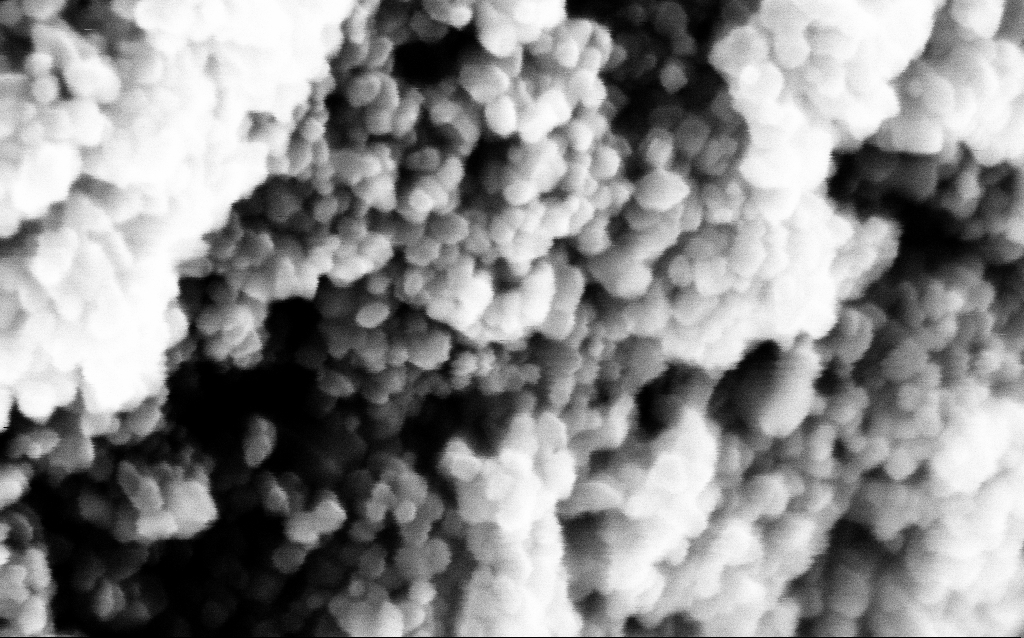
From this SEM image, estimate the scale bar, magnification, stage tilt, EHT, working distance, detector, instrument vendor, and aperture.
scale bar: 100 nm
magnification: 463.73 K X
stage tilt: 0°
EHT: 5 kV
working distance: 1.8 mm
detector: InLens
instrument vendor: Zeiss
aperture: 30 µm